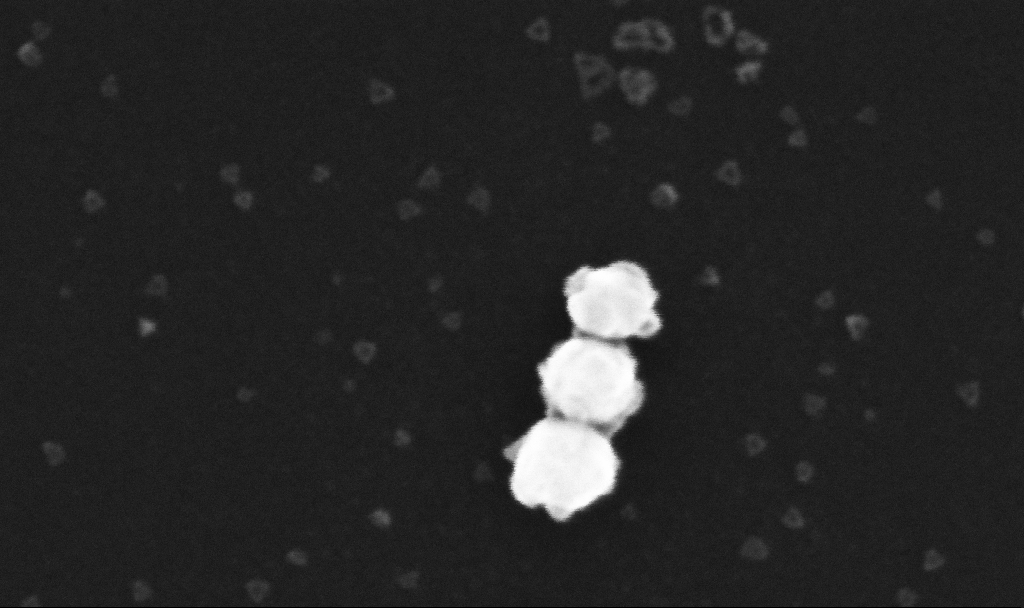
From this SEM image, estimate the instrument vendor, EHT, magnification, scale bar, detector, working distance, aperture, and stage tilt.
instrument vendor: Zeiss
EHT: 10 kV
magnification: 500 K X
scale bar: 100 nm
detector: InLens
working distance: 3.4 mm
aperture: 30 µm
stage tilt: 0°